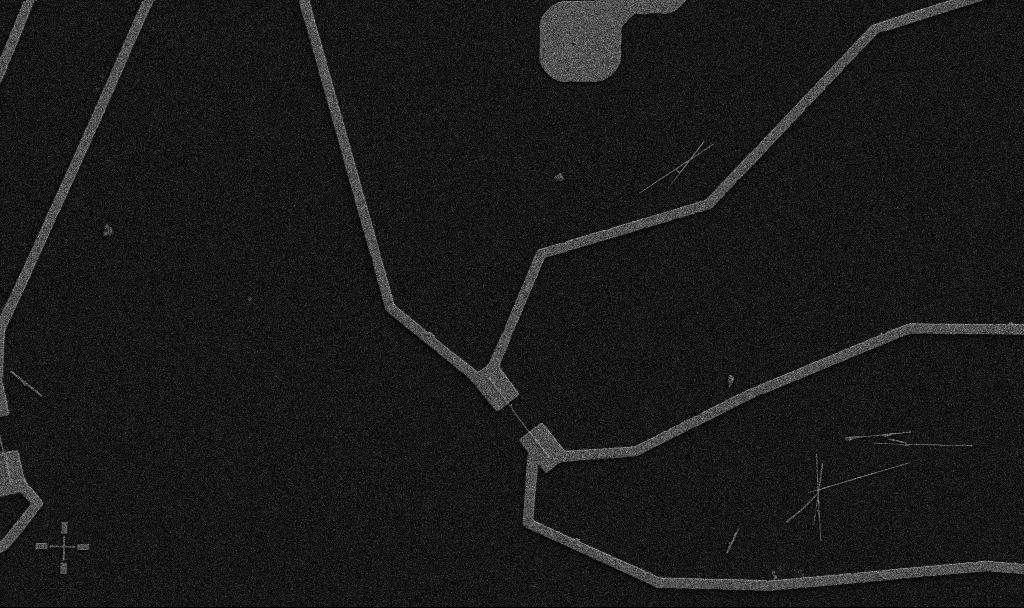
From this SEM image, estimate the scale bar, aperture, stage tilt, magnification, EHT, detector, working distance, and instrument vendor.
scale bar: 10000 nm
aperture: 30 µm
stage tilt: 0°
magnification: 5 K X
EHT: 5 kV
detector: SE2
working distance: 10.7 mm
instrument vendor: Zeiss